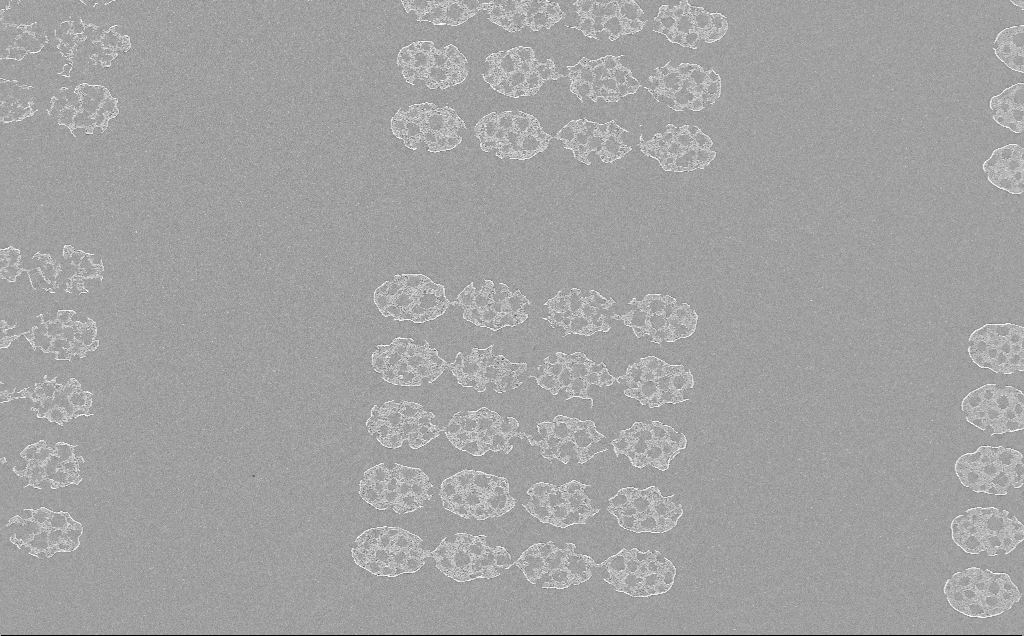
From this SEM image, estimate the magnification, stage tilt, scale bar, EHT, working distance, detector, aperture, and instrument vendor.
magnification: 3.14 K X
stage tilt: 0°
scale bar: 10000 nm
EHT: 5 kV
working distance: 6 mm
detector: InLens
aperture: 30 µm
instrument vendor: Zeiss